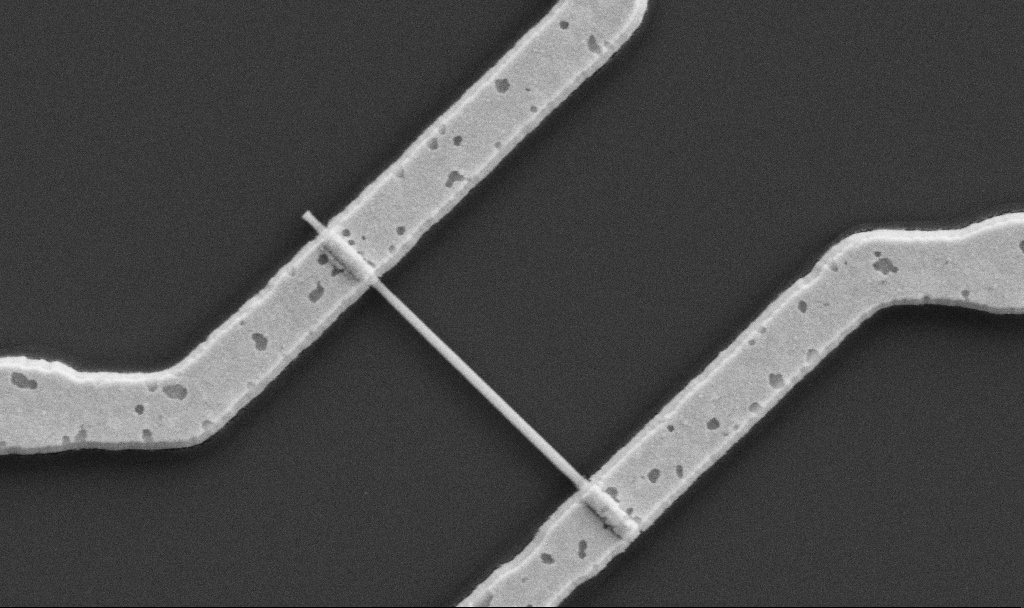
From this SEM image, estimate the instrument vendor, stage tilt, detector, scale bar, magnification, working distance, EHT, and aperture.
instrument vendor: Zeiss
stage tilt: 0°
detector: SE2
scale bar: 1000 nm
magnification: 42.43 K X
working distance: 10.7 mm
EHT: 5 kV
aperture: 30 µm